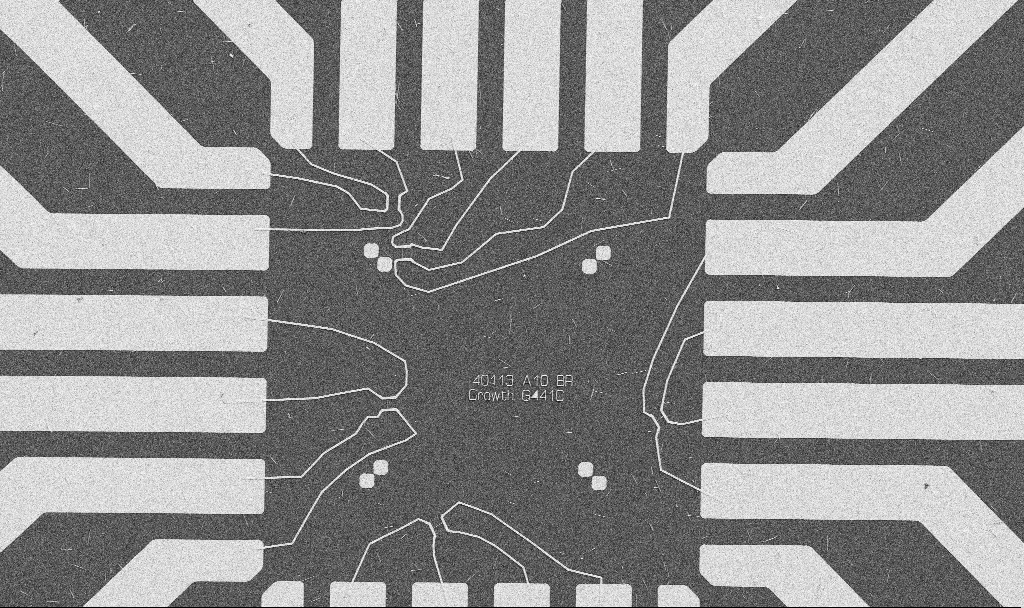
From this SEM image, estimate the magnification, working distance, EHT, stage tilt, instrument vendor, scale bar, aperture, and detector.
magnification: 1 K X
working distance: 10.7 mm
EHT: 5 kV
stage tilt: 0°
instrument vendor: Zeiss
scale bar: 20000 nm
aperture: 30 µm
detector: SE2